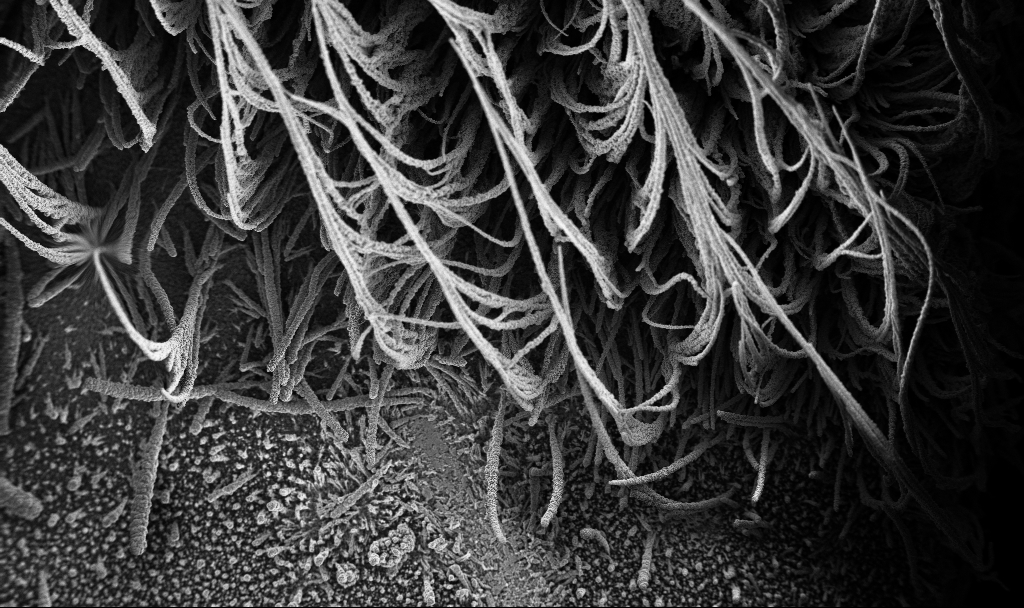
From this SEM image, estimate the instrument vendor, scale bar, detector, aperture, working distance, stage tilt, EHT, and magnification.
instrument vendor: Zeiss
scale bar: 100000 nm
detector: InLens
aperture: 30 µm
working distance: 3.7 mm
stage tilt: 0°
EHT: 3 kV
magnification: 0.15 K X